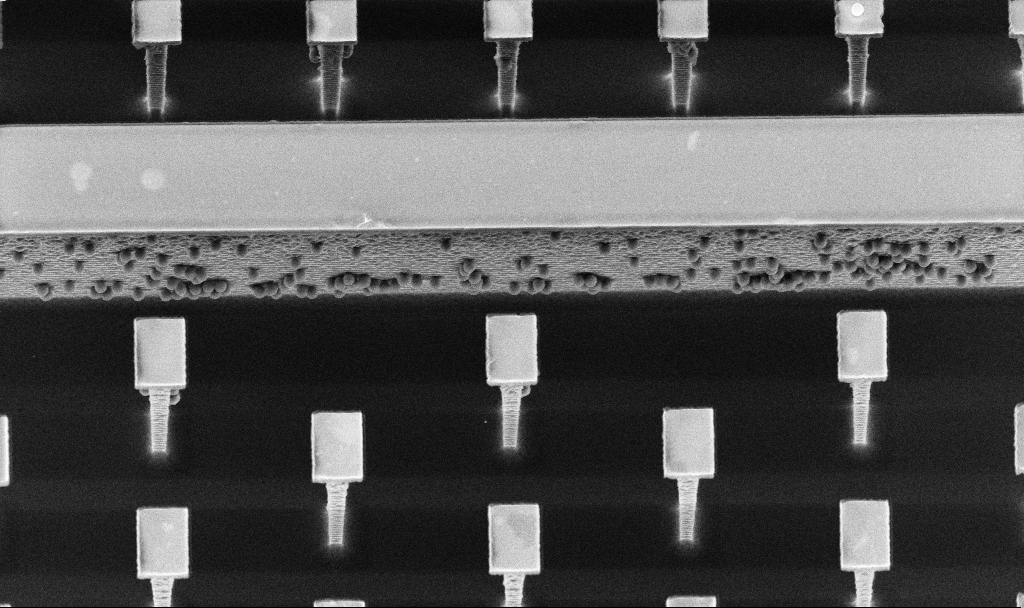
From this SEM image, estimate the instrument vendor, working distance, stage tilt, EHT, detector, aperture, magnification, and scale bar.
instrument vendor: Zeiss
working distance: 4.5 mm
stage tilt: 20°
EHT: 5 kV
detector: InLens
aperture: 30 µm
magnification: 6.19 K X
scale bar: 10000 nm